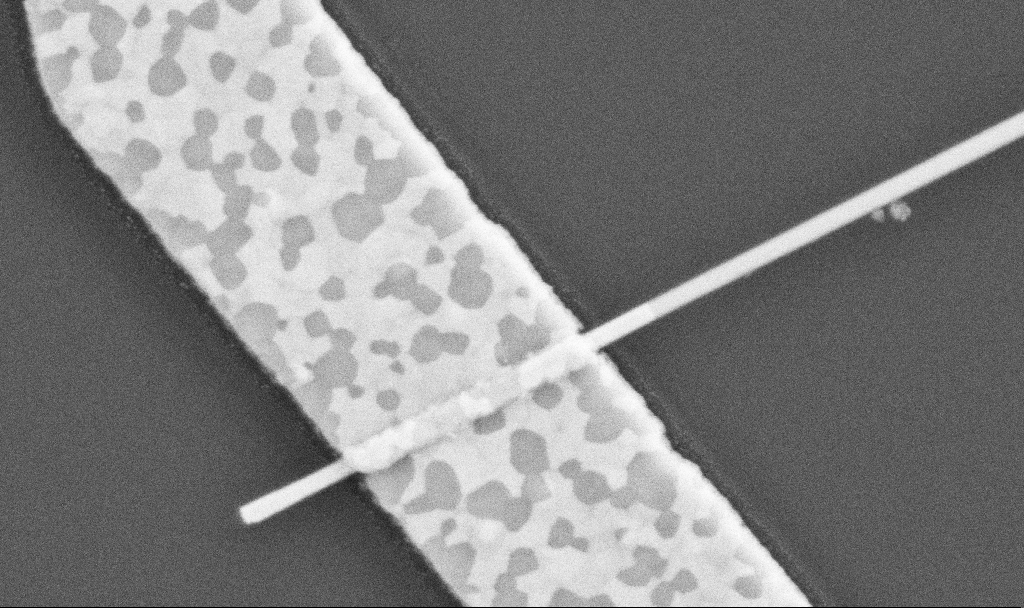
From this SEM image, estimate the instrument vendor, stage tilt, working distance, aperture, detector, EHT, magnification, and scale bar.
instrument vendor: Zeiss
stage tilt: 0°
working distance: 10.5 mm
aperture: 30 µm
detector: SE2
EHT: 5 kV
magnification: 100 K X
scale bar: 200 nm